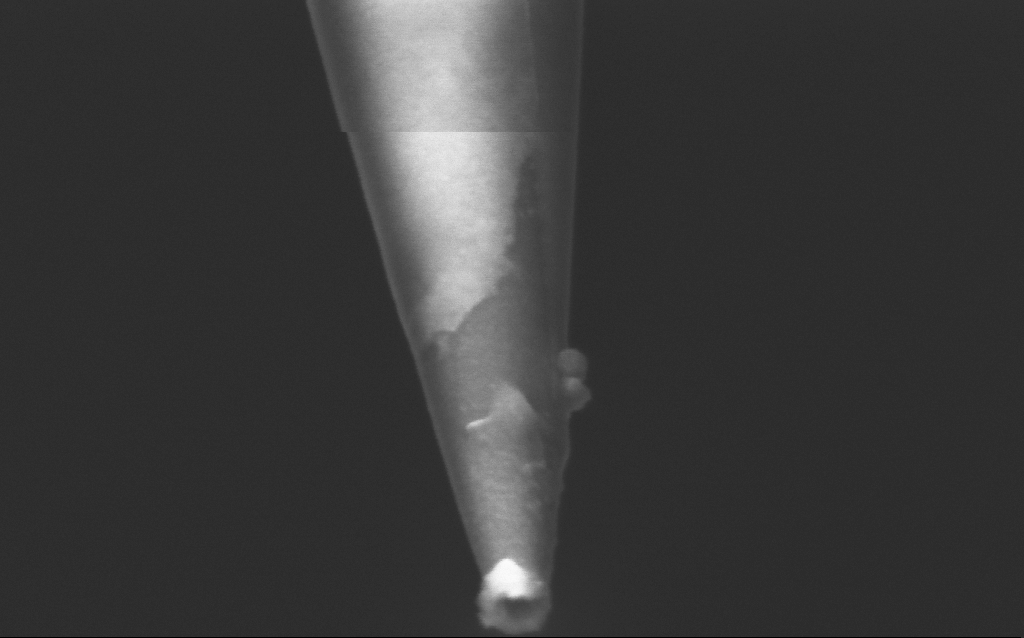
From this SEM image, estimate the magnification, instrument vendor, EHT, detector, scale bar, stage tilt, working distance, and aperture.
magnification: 250 K X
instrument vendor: Zeiss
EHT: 2.5 kV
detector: InLens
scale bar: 200 nm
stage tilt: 45°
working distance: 5 mm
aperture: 30 µm